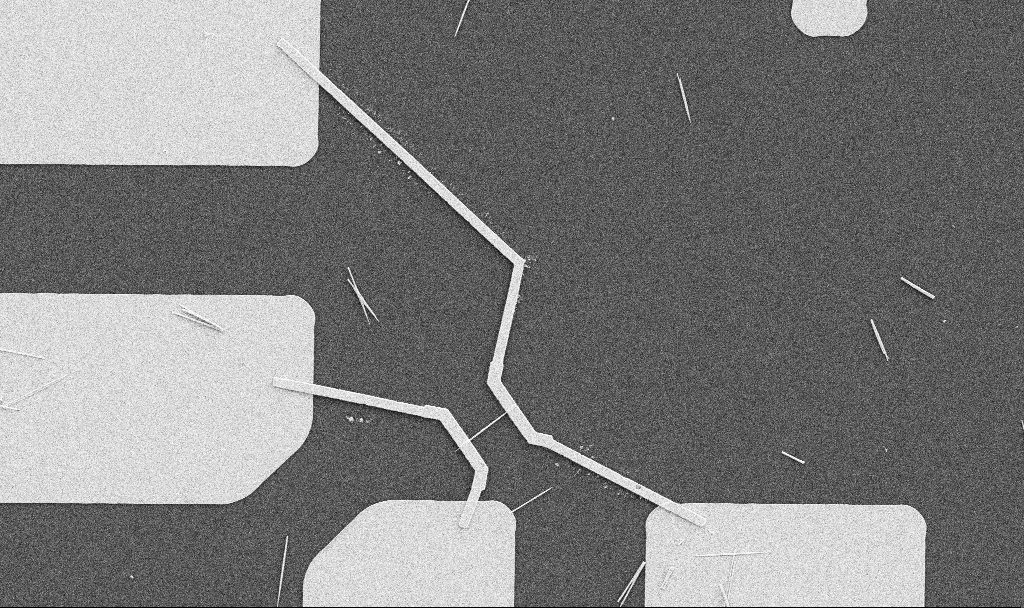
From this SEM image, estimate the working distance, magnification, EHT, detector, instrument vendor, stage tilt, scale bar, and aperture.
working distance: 10.7 mm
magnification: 5 K X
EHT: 5 kV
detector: SE2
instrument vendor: Zeiss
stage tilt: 0°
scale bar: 10000 nm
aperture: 30 µm